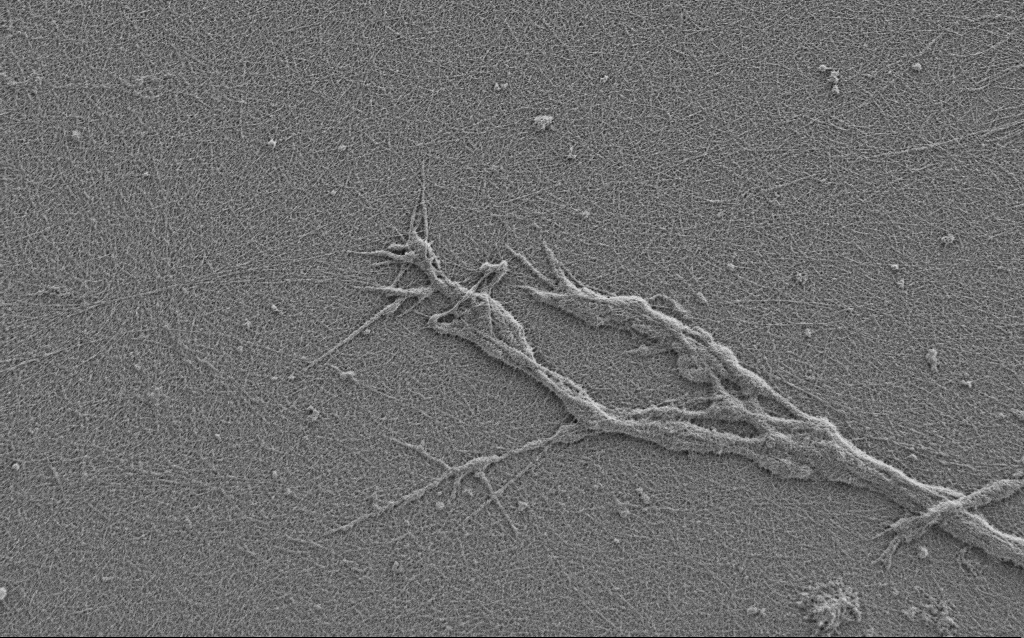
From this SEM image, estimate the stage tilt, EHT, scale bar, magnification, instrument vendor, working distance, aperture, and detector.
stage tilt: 0°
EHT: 0.9 kV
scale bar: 2000 nm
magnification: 7.5 K X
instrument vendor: Zeiss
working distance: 4 mm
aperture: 30 µm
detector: SE2